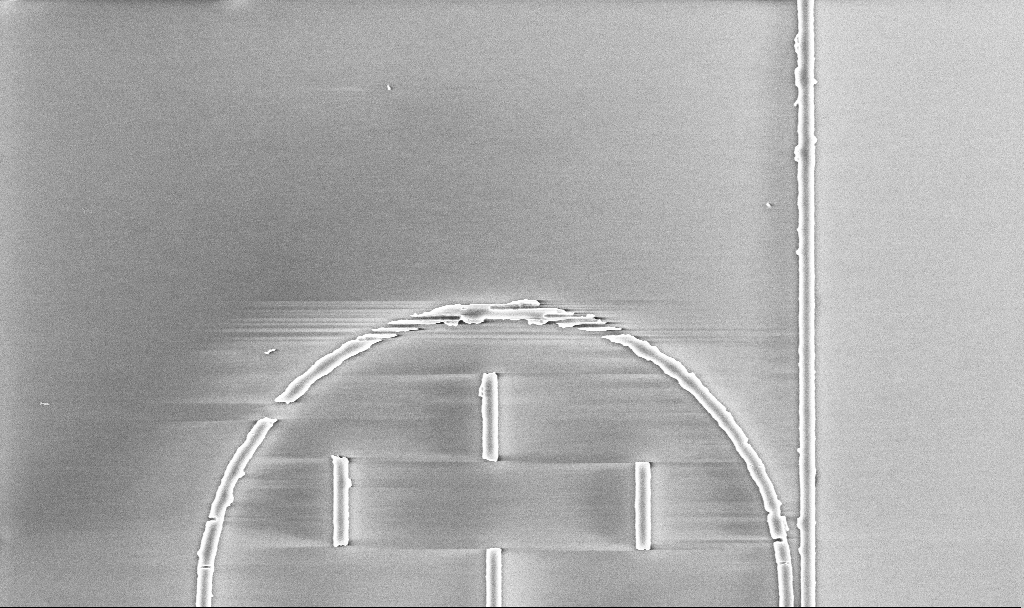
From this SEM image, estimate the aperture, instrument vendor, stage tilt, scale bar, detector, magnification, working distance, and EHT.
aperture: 30 µm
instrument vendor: Zeiss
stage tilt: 0°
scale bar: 2000 nm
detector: InLens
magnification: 11.07 K X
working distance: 5.2 mm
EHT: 5 kV